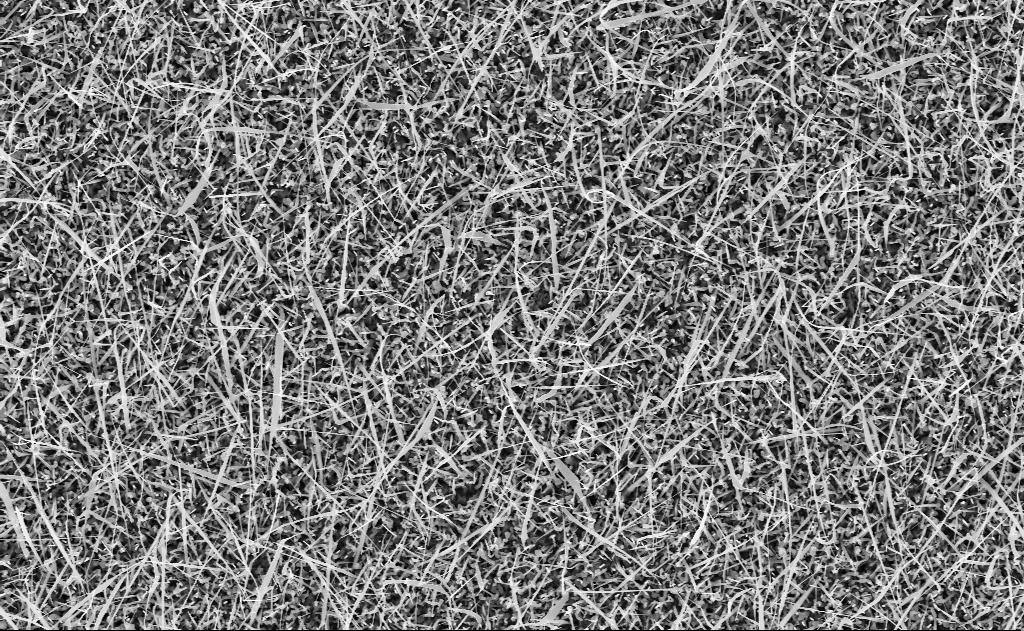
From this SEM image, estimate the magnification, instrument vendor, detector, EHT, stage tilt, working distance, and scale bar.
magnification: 10 K X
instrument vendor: Zeiss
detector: InLens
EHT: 10 kV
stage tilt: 0°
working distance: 10 mm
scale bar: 2000 nm